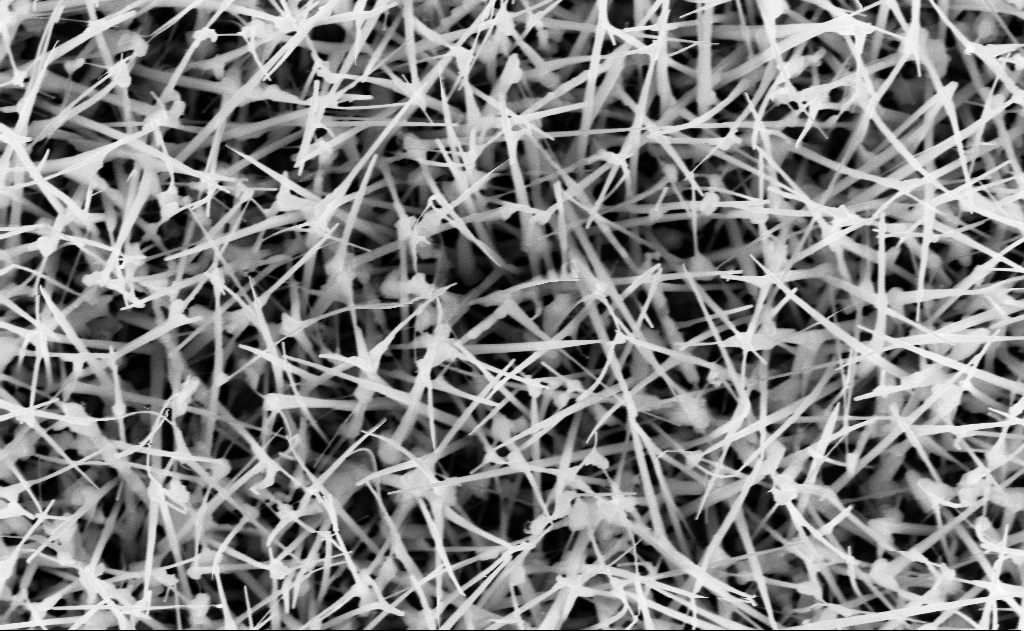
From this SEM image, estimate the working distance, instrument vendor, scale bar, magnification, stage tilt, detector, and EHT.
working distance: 14 mm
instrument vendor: Zeiss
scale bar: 1000 nm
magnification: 40 K X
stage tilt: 0°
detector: InLens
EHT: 10 kV